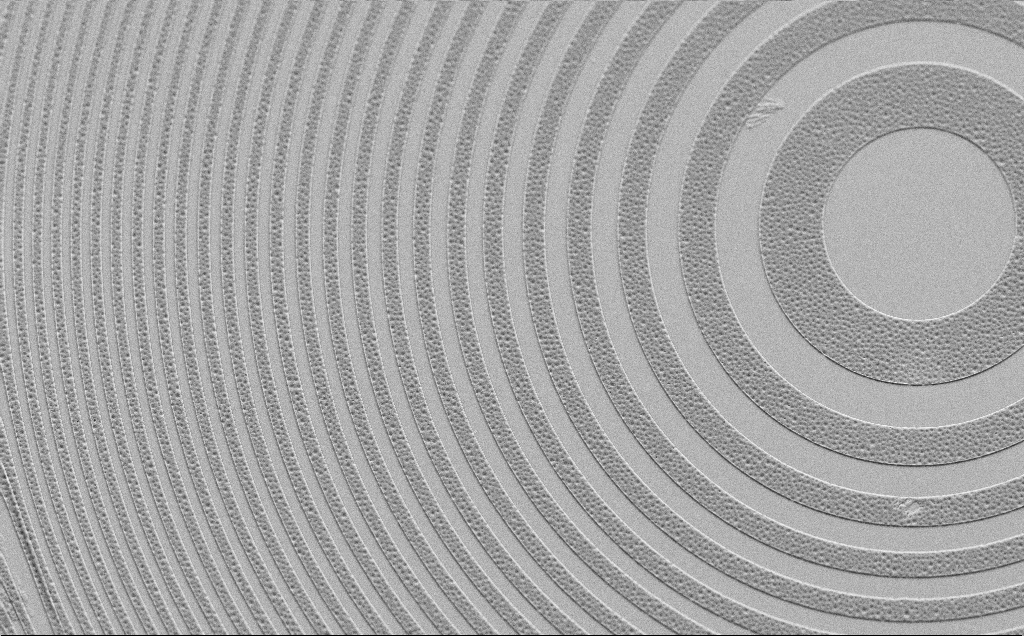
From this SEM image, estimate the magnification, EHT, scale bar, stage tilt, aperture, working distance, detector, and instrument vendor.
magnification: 2.39 K X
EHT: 5 kV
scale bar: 10000 nm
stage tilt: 45°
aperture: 30 µm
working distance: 7 mm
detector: SE2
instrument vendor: Zeiss